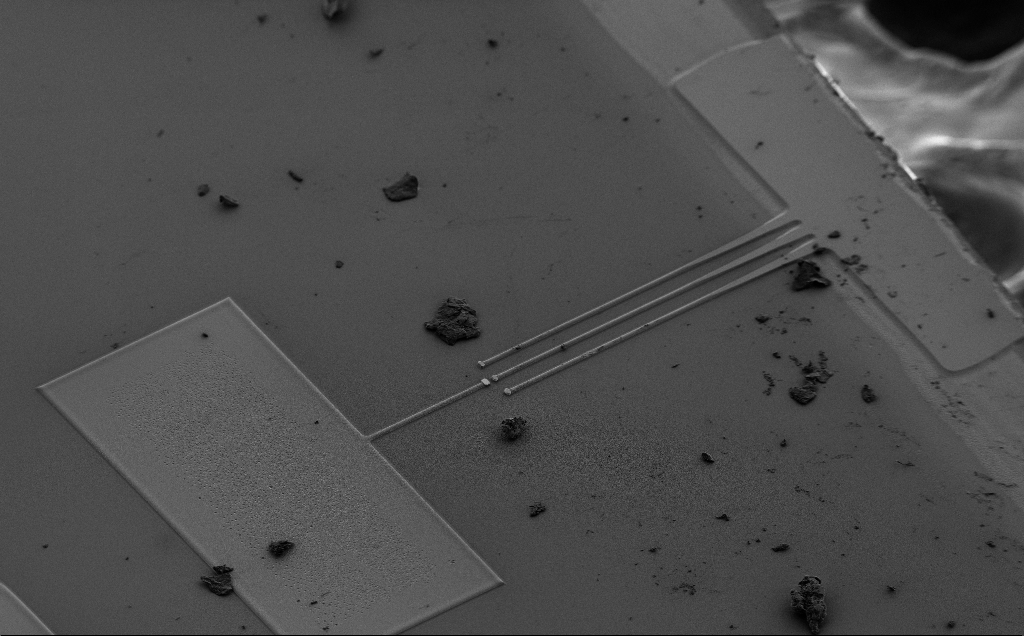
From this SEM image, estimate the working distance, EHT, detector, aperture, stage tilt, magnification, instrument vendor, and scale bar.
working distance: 10 mm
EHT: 2 kV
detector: SE2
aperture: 30 µm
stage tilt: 43°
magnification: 0.427 K X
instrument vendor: Zeiss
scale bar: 100000 nm